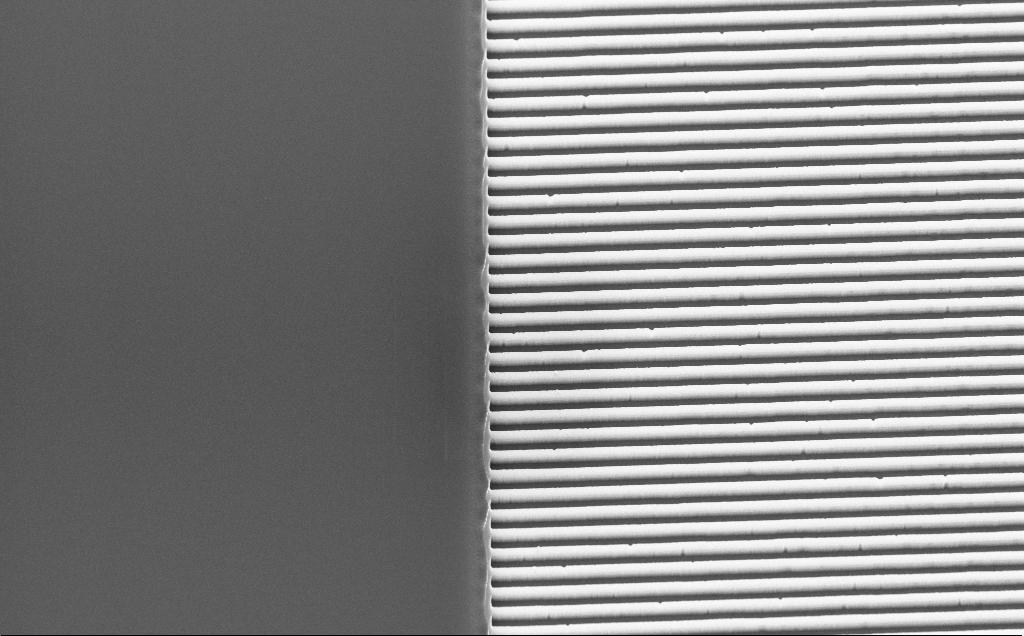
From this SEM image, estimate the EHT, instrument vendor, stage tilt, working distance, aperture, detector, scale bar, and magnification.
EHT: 10 kV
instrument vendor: Zeiss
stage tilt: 30°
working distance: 4 mm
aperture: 30 µm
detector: InLens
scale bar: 1000 nm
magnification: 14.04 K X